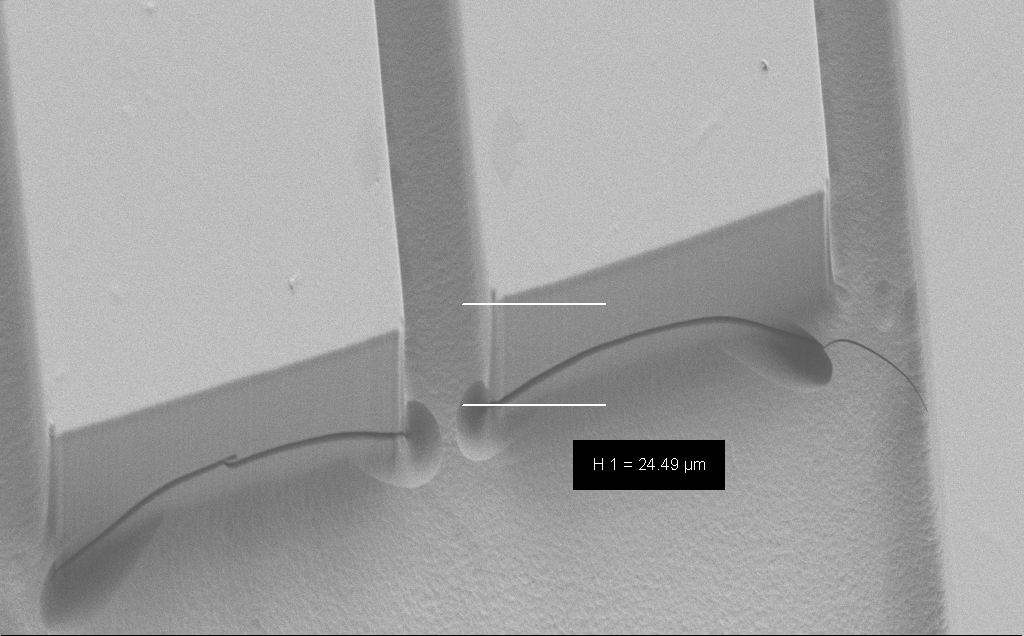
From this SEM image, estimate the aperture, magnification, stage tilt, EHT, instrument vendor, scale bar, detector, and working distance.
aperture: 30 µm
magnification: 1.51 K X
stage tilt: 30°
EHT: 1.2 kV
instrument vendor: Zeiss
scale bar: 20000 nm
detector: SE2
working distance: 7 mm